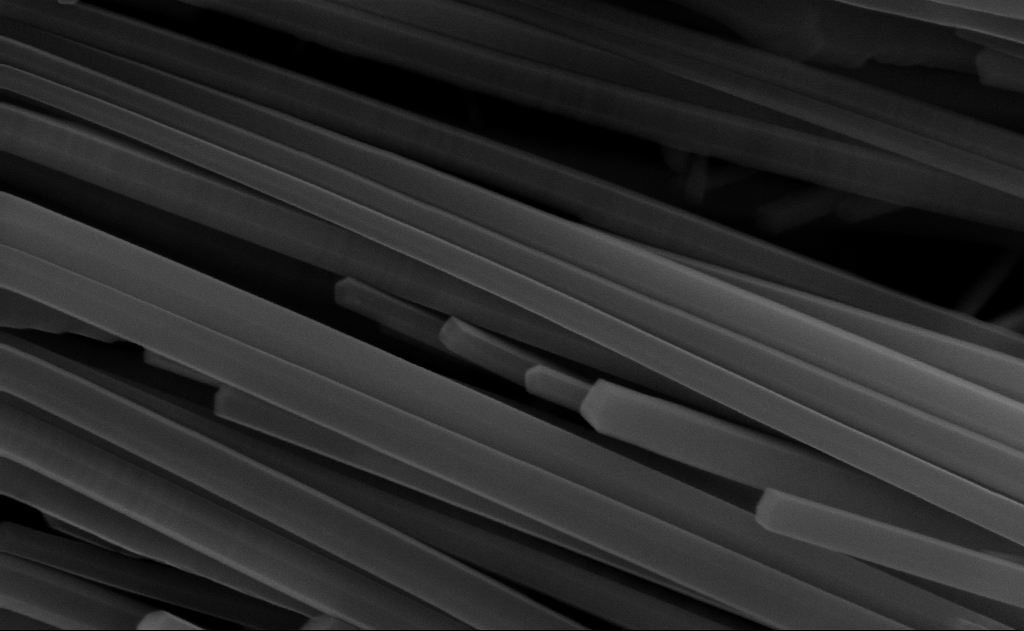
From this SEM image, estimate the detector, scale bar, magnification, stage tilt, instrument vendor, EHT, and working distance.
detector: InLens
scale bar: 200 nm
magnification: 150 K X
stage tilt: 0°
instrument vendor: Zeiss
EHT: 10 kV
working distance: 9 mm